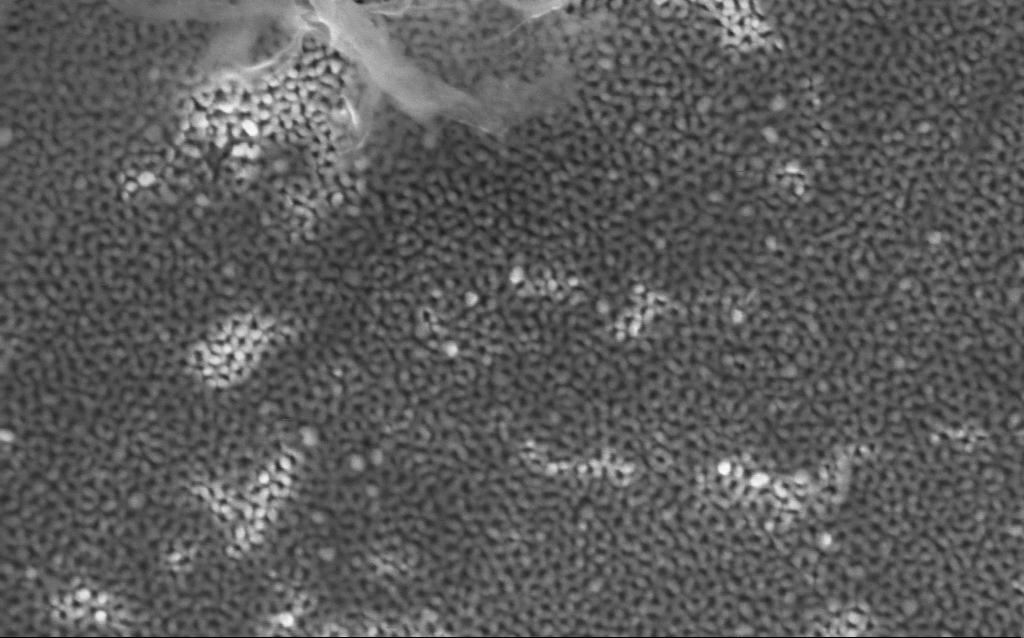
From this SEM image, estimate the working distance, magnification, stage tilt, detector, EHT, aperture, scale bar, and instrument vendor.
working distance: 4.1 mm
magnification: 160.72 K X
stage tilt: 0.1°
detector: InLens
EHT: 5 kV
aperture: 30 µm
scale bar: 100 nm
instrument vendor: Zeiss